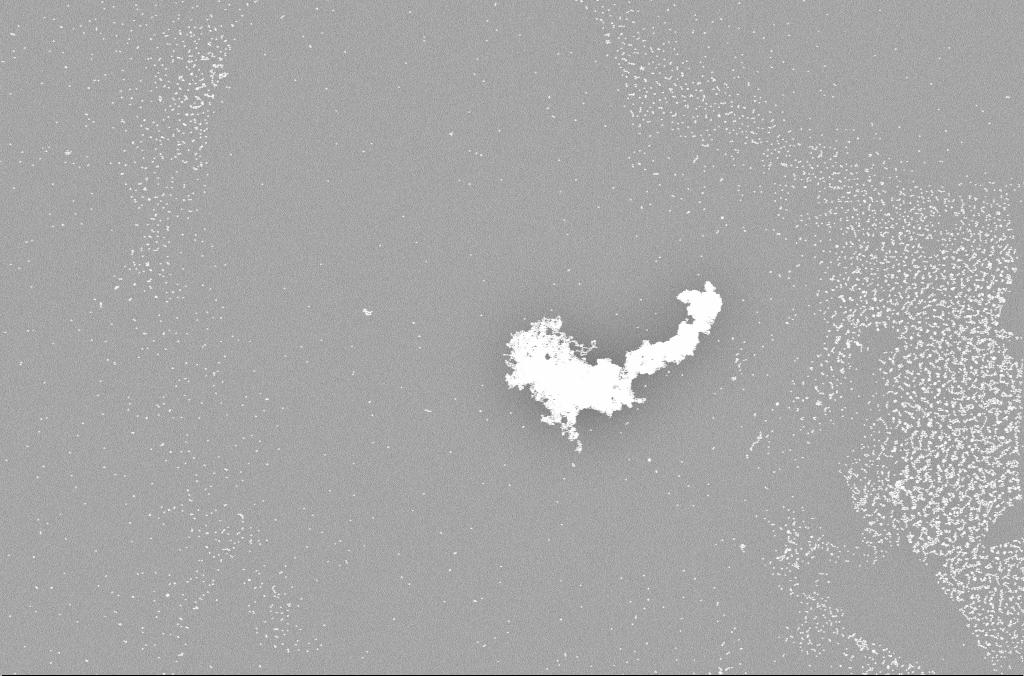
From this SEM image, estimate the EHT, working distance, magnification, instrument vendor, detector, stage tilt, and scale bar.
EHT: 10 kV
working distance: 4.9 mm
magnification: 36.38 K X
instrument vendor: Zeiss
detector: HDAsB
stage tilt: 0°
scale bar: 1000 nm